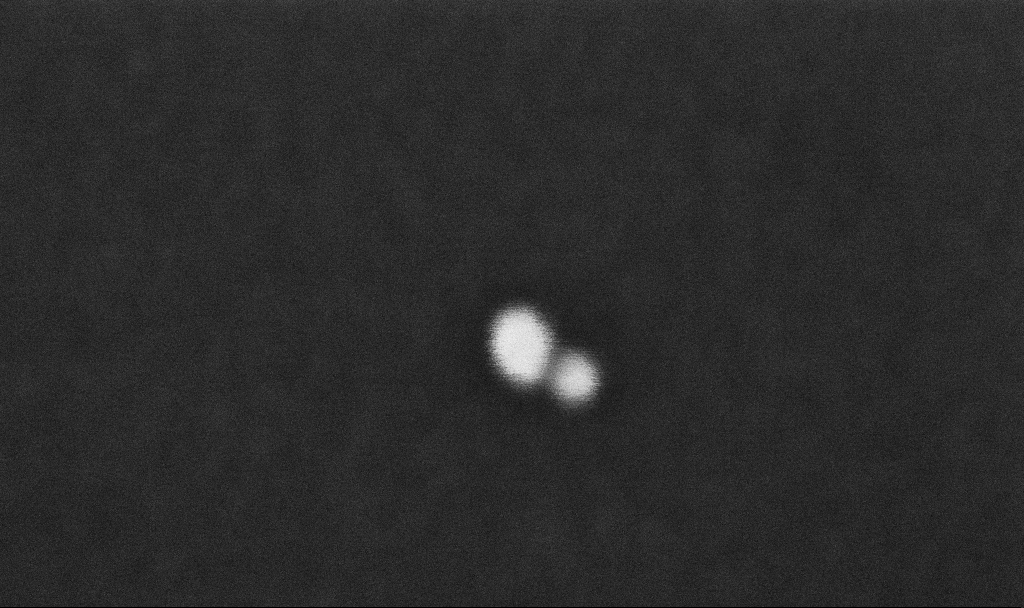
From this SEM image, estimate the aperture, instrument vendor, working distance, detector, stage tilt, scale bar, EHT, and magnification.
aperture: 30 µm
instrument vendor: Zeiss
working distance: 4.3 mm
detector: InLens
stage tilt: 0°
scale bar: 20 nm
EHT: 10 kV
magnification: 1412.32 K X